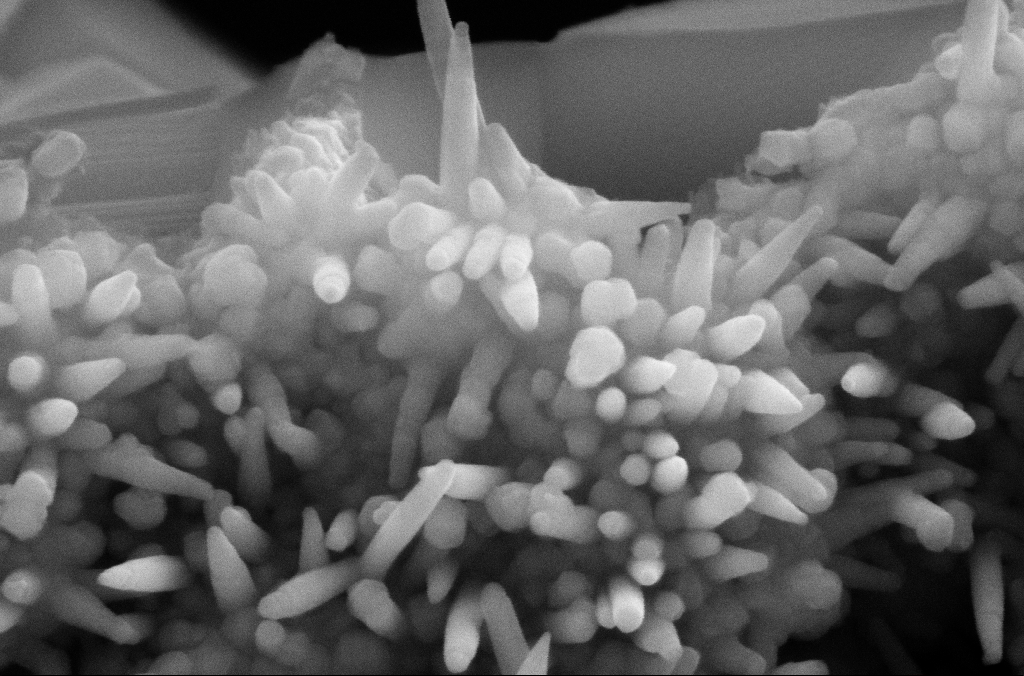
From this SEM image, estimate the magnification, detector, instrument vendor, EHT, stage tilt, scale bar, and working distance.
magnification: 226.13 K X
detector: SE2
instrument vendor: Zeiss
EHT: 10 kV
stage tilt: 0.1°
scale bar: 200 nm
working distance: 5.2 mm